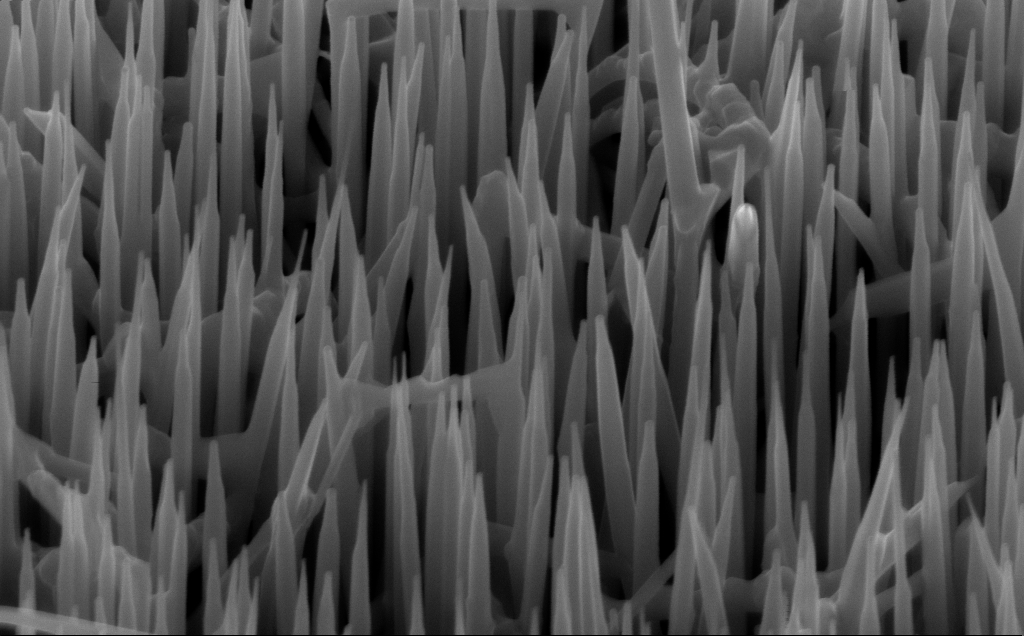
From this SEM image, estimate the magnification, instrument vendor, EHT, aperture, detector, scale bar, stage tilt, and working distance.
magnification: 80 K X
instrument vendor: Zeiss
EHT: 10 kV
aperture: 30 µm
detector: InLens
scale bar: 200 nm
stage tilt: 45°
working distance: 6 mm